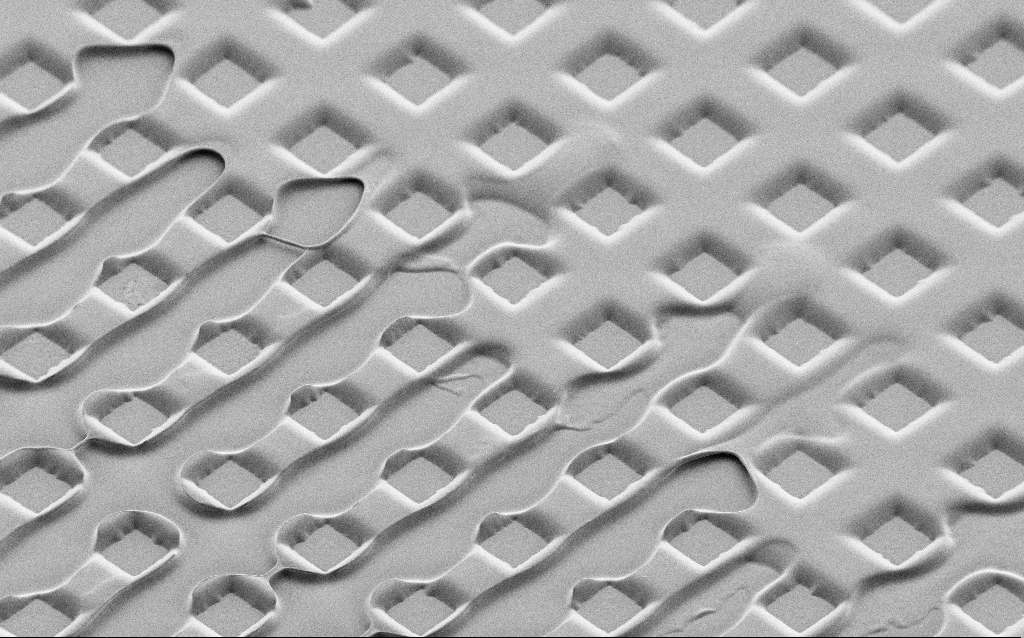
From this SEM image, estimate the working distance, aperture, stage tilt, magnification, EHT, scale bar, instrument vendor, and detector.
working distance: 6 mm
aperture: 30 µm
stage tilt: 45°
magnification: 0.345 K X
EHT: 1.5 kV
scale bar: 100000 nm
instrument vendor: Zeiss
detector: SE2